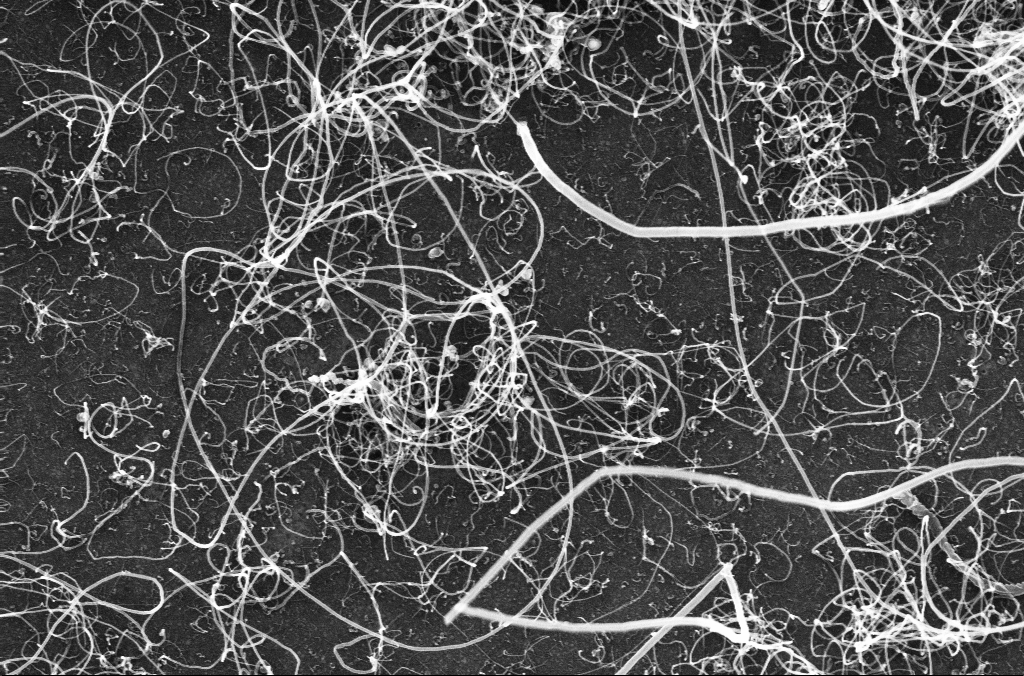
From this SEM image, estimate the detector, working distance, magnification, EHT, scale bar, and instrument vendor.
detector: InLens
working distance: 3.3 mm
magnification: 50 K X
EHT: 10 kV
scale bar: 1000 nm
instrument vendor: Zeiss